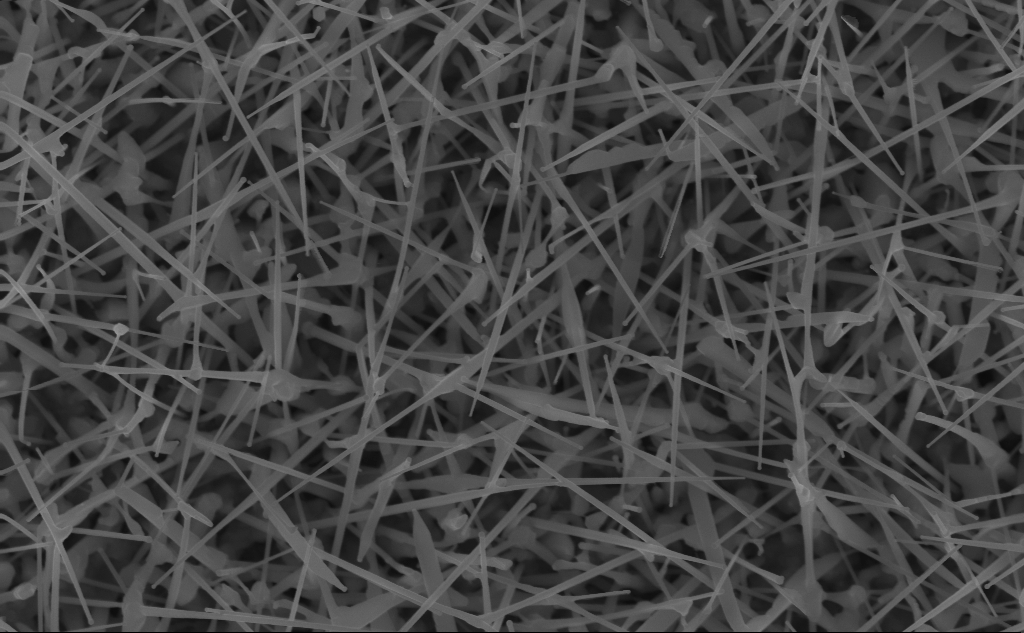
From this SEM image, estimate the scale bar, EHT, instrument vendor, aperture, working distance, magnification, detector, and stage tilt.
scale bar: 1000 nm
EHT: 10 kV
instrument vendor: Zeiss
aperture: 30 µm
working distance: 5 mm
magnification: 40 K X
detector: InLens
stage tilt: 0°